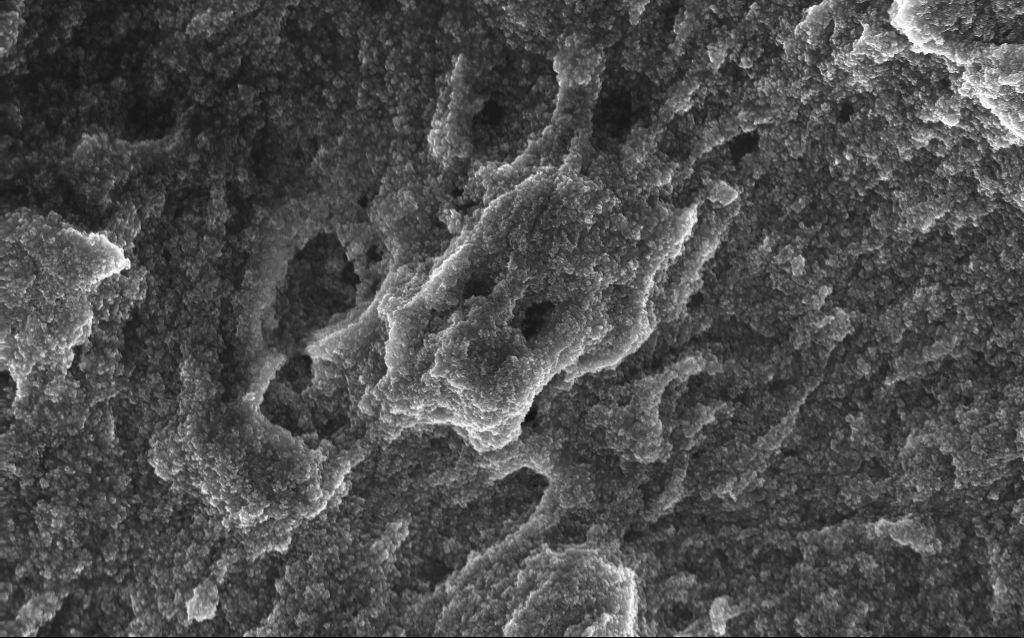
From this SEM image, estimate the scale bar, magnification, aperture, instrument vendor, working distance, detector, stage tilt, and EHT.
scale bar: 1000 nm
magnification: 20.87 K X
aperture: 30 µm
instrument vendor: Zeiss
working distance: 2.6 mm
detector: InLens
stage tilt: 0°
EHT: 10 kV